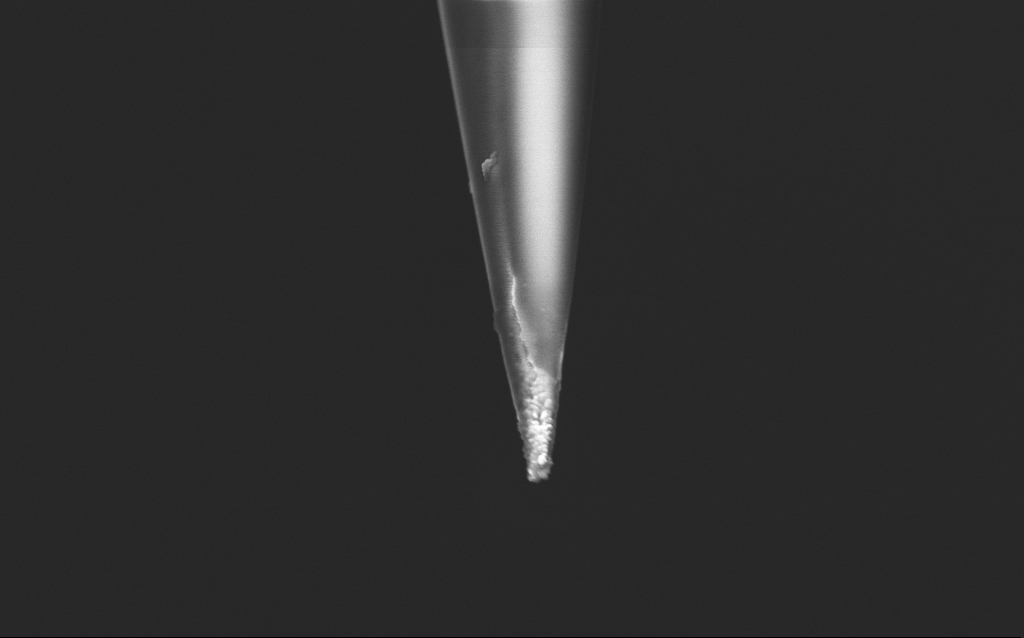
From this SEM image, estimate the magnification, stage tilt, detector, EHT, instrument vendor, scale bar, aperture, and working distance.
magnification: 50 K X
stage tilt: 45°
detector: InLens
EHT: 2.5 kV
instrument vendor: Zeiss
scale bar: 1000 nm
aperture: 30 µm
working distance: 5 mm